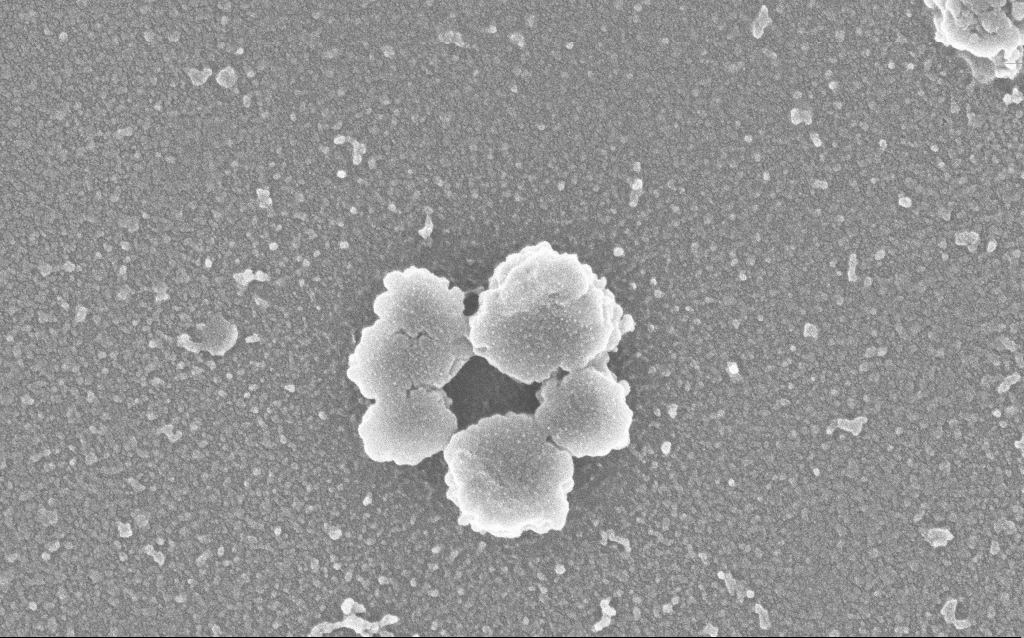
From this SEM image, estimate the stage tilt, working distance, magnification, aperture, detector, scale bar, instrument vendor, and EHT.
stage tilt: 0°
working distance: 2.5 mm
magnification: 100 K X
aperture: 30 µm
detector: InLens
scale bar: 200 nm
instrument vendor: Zeiss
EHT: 3 kV